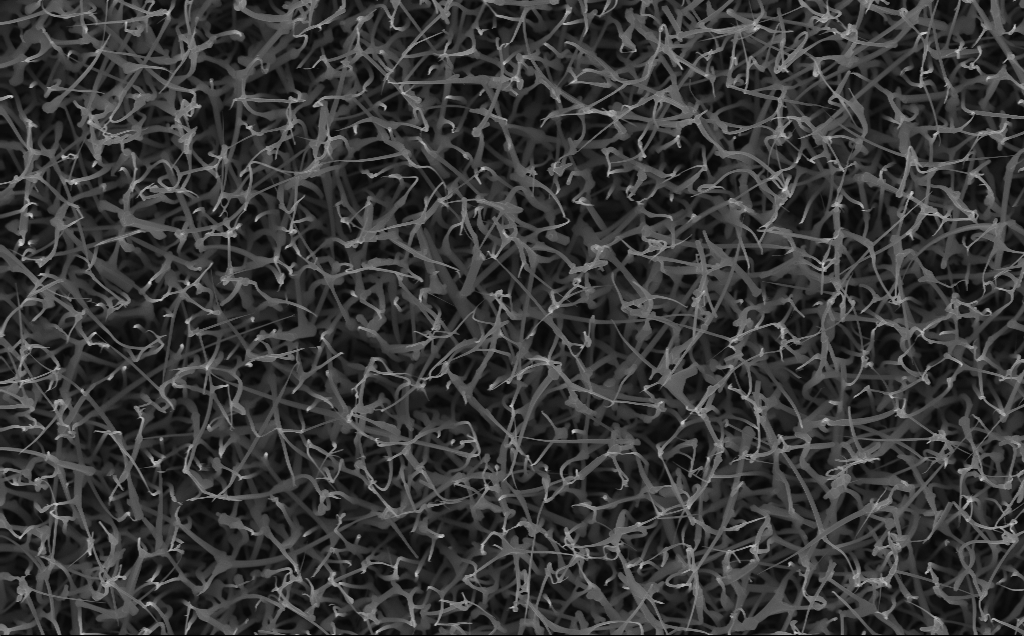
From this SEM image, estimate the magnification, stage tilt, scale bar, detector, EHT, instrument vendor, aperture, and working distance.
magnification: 20 K X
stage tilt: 0°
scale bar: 1000 nm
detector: InLens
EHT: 10 kV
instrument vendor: Zeiss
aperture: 30 µm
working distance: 5 mm